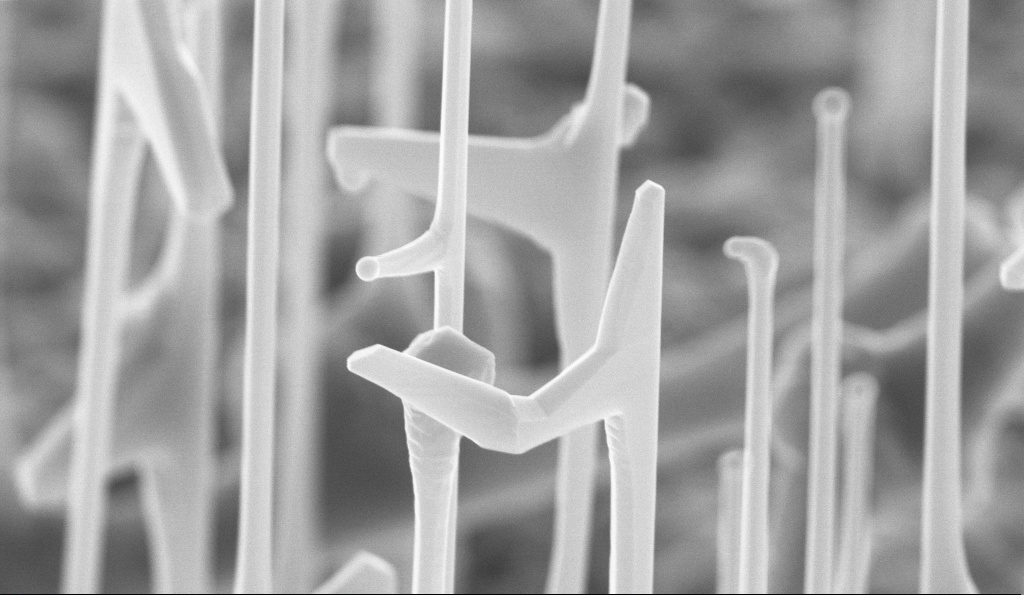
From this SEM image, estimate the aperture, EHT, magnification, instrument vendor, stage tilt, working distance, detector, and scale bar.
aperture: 30 µm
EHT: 10 kV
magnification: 50.17 K X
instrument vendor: Zeiss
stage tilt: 45°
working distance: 3.6 mm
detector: InLens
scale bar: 1000 nm